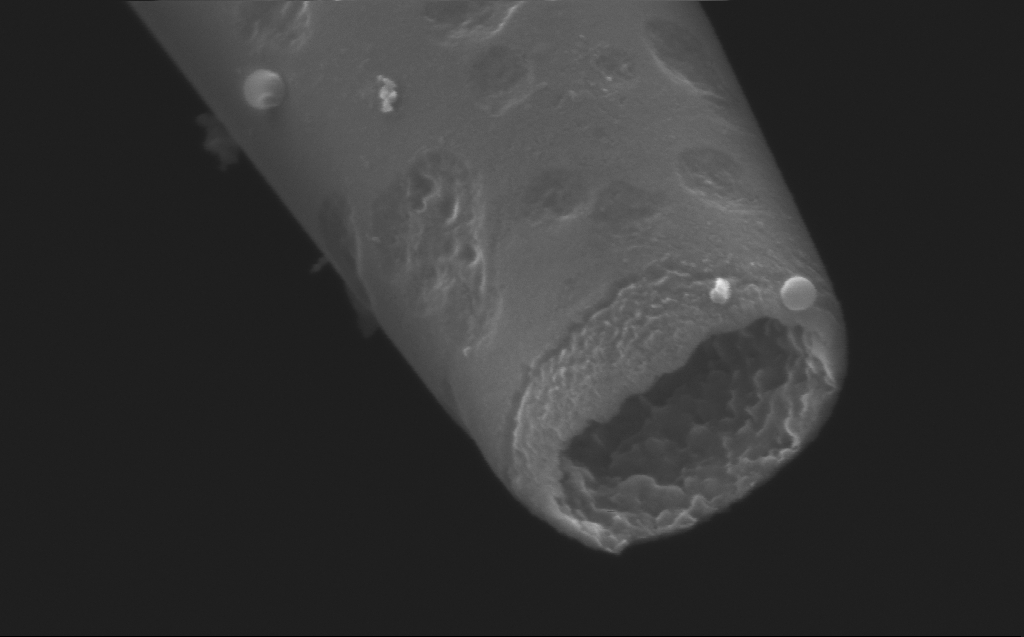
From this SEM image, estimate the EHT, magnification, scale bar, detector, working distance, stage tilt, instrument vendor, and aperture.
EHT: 5 kV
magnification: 178.04 K X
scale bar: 200 nm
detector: InLens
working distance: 4 mm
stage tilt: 45°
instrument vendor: Zeiss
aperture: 30 µm